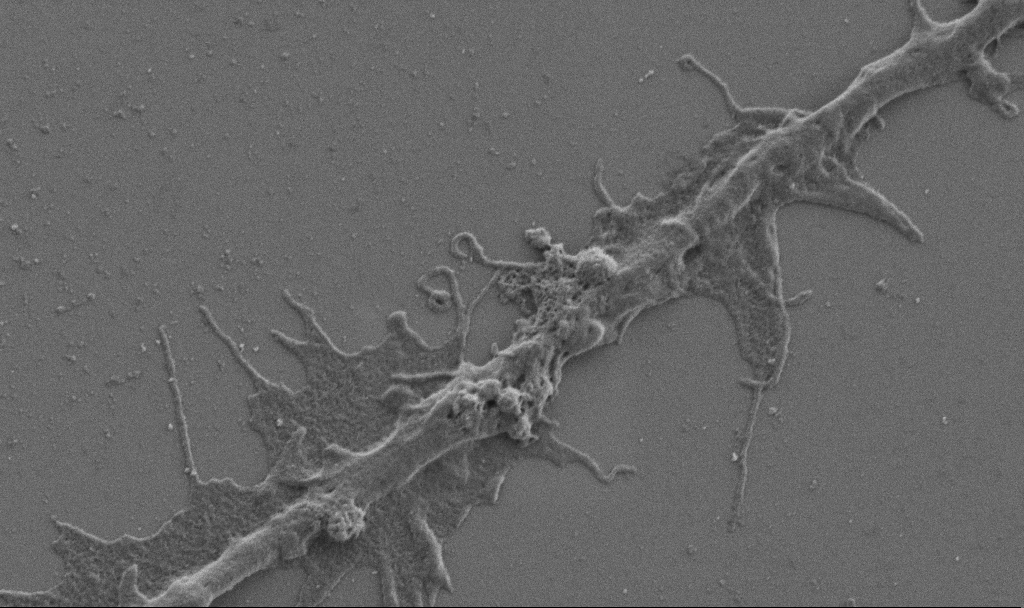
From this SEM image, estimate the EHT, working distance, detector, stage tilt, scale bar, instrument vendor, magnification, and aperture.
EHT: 1 kV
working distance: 6.9 mm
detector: SE2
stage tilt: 0°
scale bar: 2000 nm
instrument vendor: Zeiss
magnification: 20 K X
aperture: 30 µm